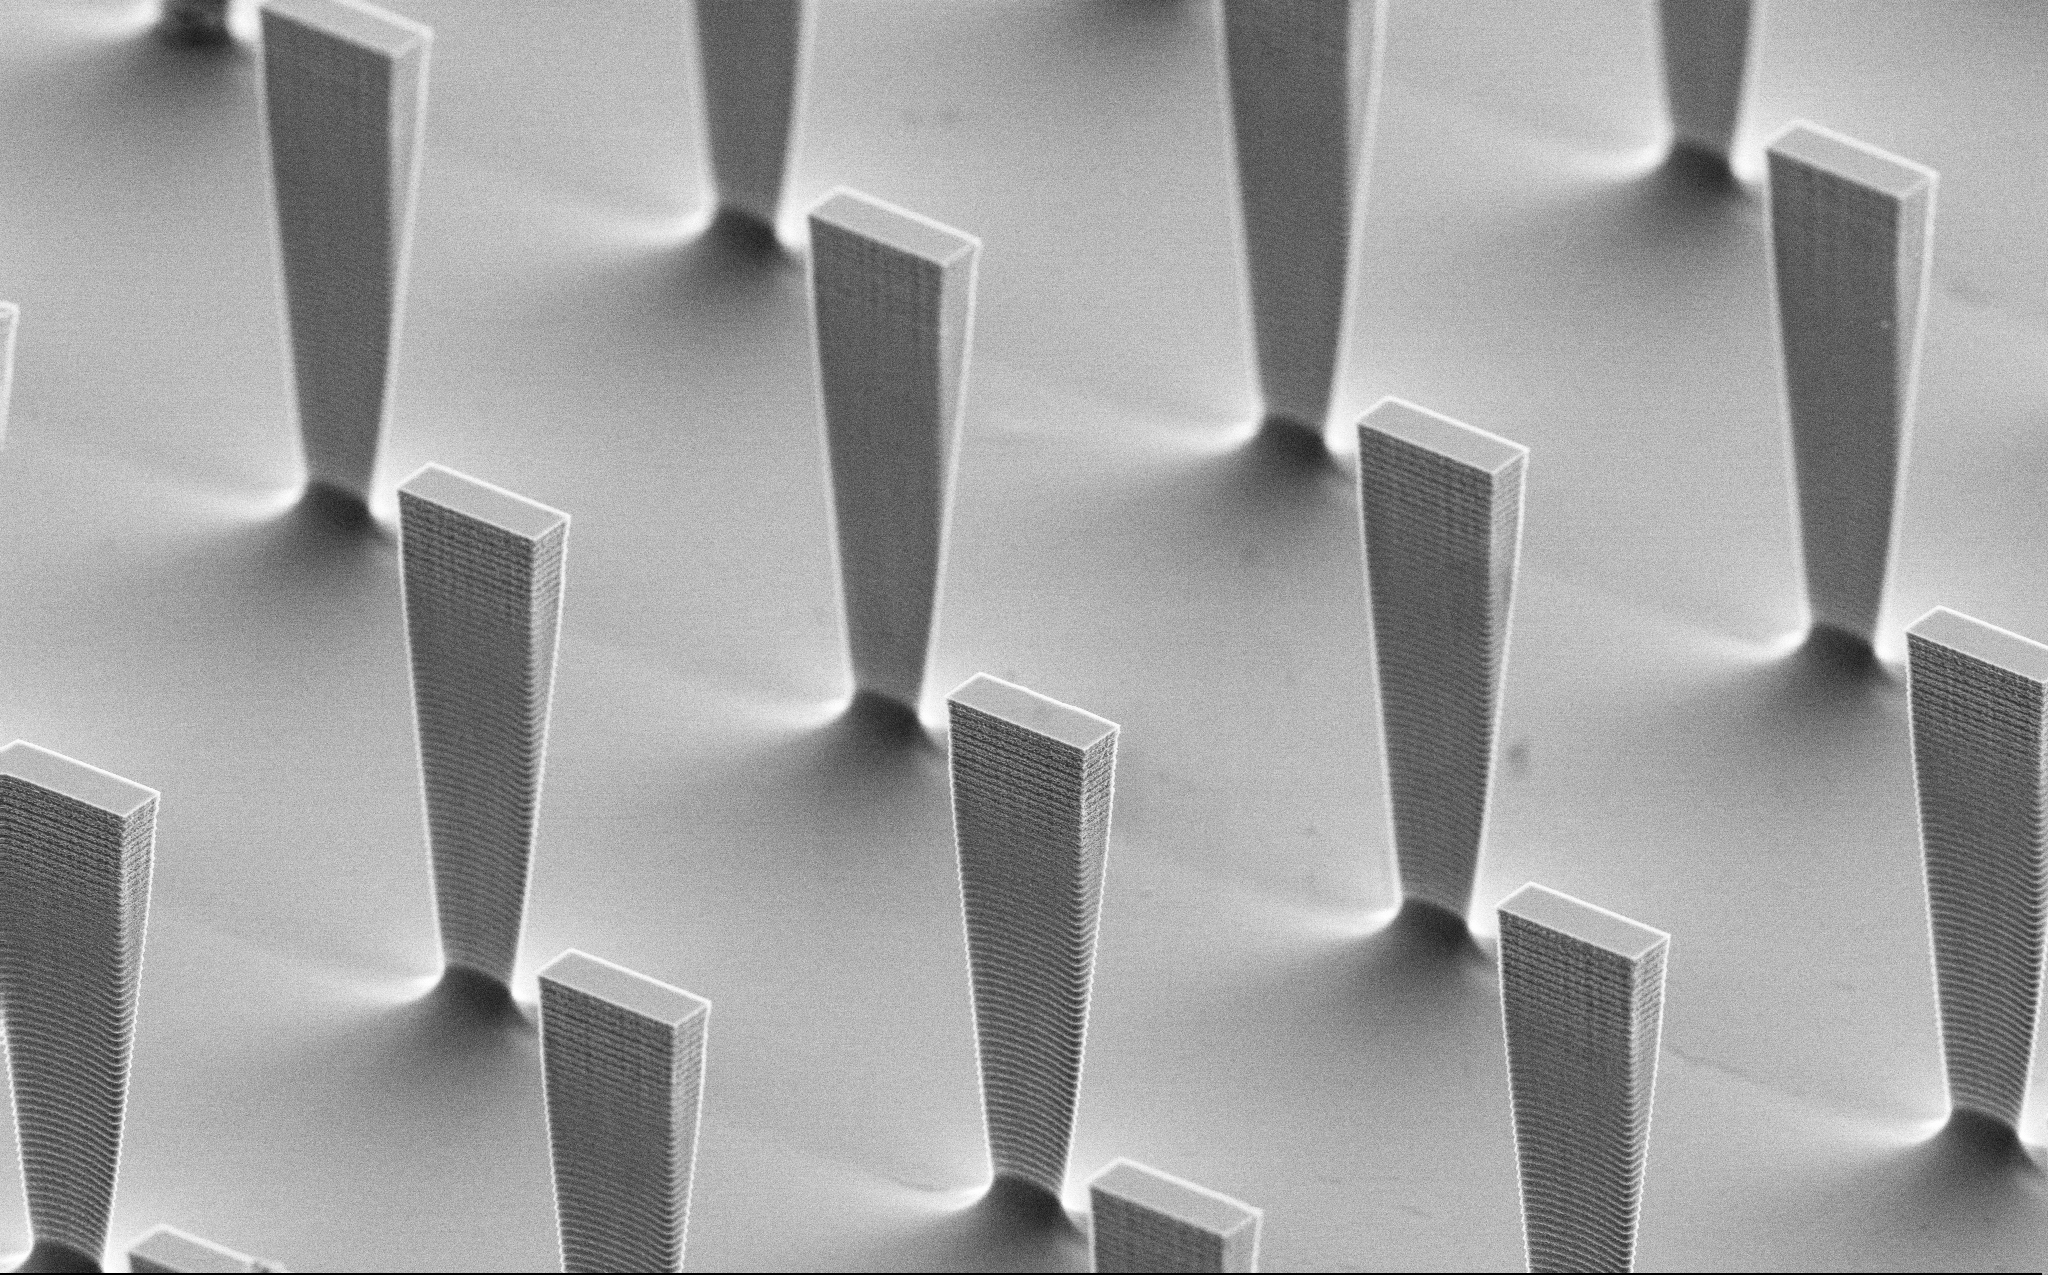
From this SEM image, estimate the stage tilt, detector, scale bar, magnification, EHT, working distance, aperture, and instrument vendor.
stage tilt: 60°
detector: SE2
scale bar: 10000 nm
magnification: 4.21 K X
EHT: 5 kV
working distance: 9.7 mm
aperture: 30 µm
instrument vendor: Zeiss